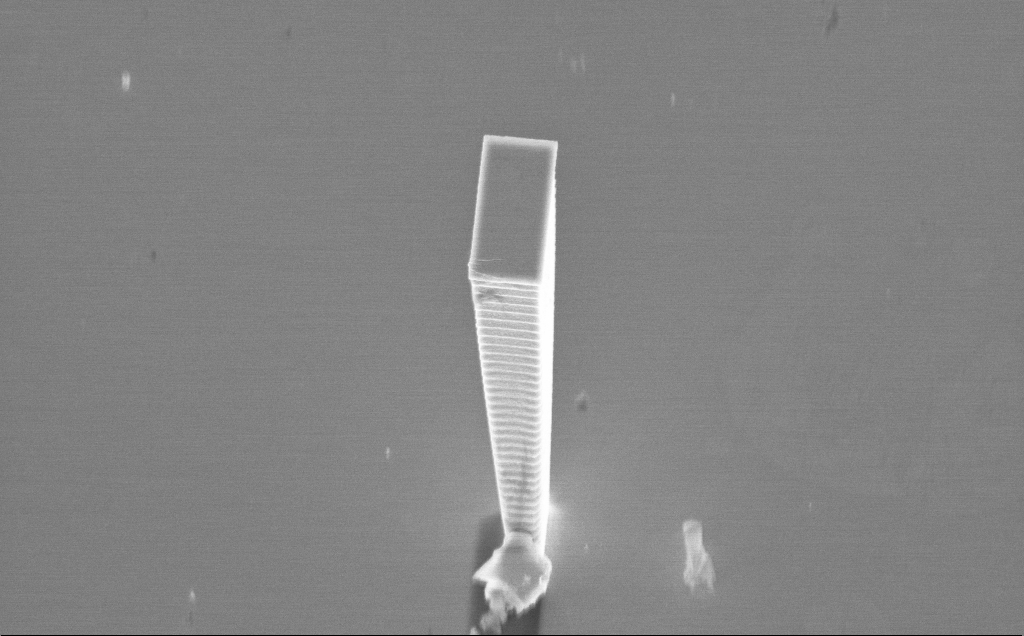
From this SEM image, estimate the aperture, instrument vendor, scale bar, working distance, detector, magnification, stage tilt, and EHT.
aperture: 30 µm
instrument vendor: Zeiss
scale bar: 2000 nm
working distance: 3 mm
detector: InLens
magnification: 9.71 K X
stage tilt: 36.8°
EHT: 5 kV